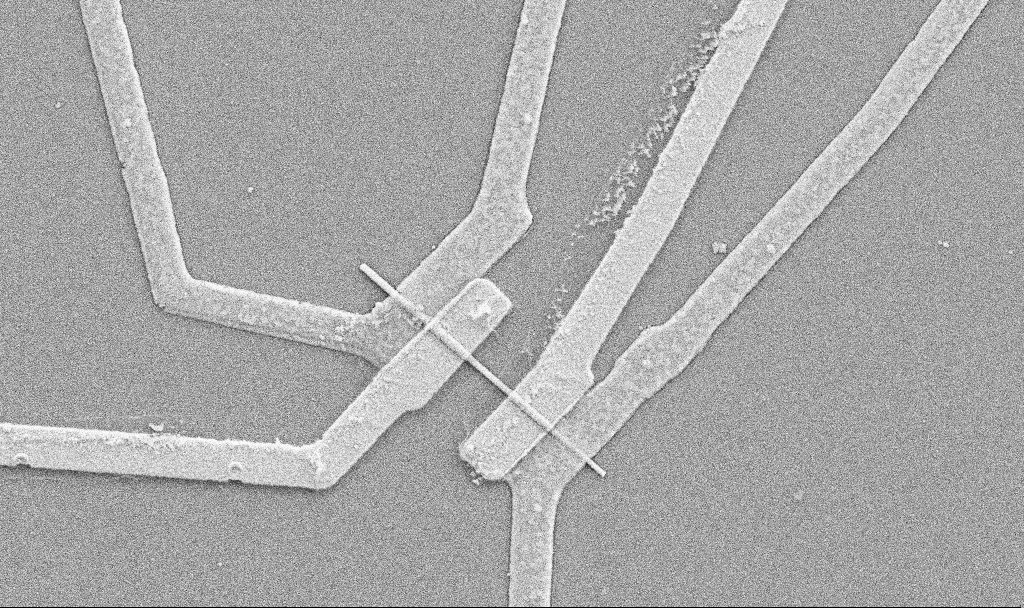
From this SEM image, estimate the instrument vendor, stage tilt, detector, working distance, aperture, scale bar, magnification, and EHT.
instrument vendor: Zeiss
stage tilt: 0°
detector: SE2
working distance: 10.7 mm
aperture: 30 µm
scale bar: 1000 nm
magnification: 20 K X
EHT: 5 kV